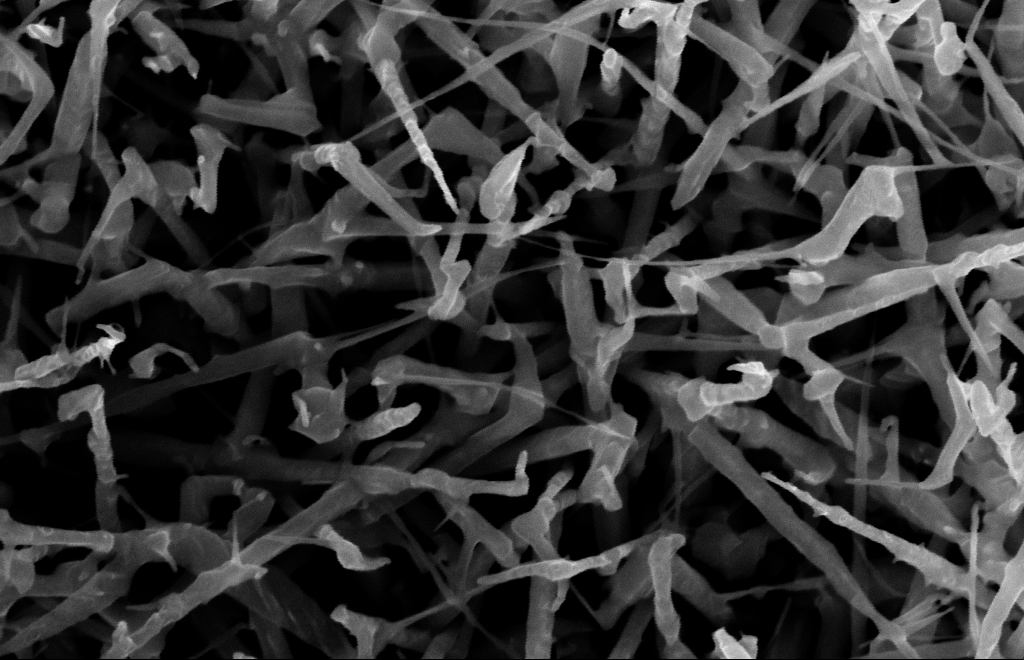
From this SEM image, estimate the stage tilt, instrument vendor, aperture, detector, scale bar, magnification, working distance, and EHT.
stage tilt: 0°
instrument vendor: Zeiss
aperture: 30 µm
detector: InLens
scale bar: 100 nm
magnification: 80 K X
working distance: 15 mm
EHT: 10 kV